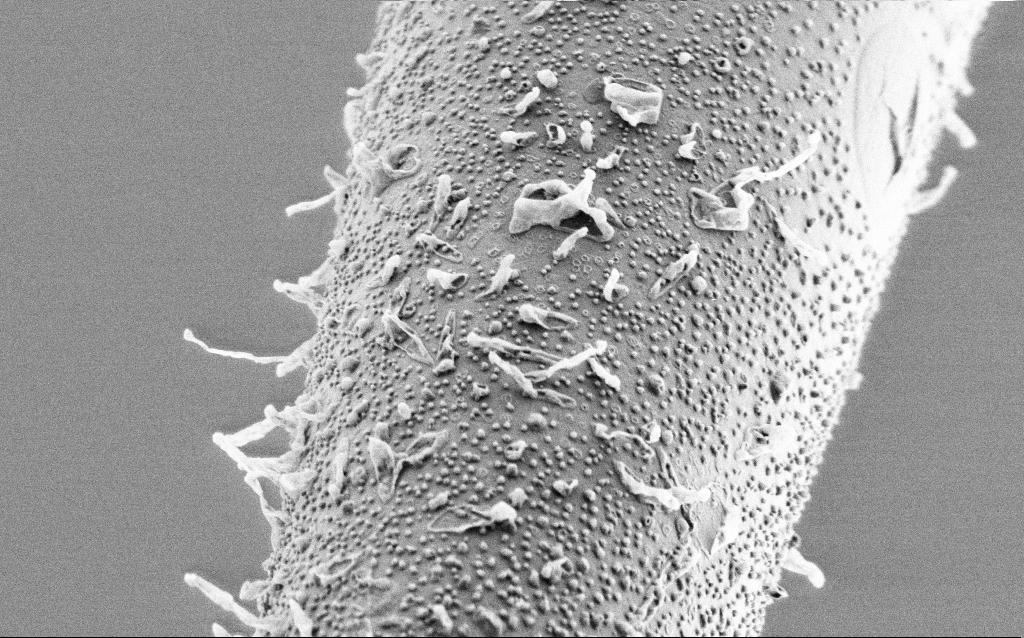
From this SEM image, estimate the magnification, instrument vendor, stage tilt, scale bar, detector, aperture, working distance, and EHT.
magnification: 25 K X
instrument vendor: Zeiss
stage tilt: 45°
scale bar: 1000 nm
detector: SE2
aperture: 30 µm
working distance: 6.6 mm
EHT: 1 kV